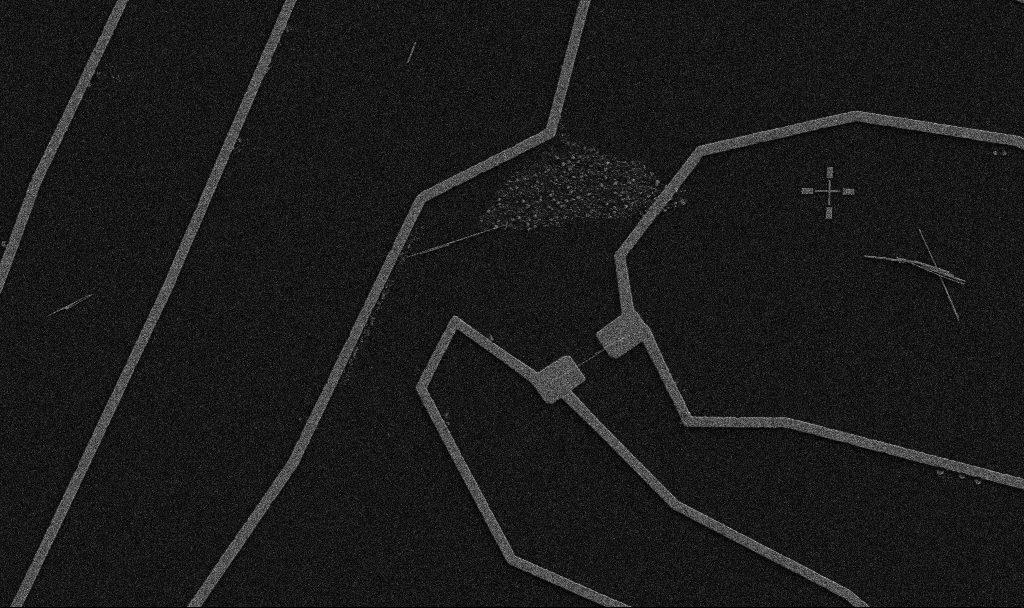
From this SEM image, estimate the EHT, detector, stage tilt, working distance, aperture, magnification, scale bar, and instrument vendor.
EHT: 5 kV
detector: SE2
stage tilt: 0°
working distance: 10.7 mm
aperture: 30 µm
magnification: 5 K X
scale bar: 10000 nm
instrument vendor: Zeiss